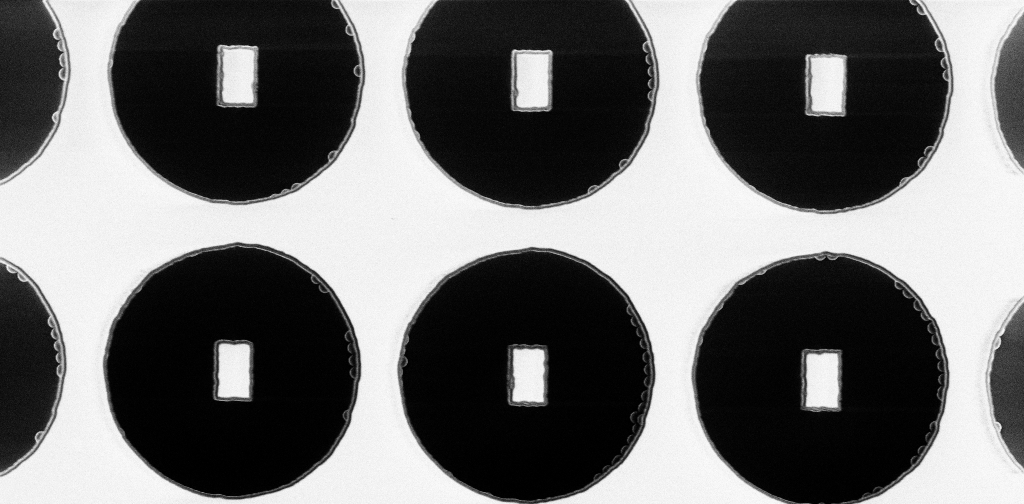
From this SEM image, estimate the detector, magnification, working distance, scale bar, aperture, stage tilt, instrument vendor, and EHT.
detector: InLens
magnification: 7.65 K X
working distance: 5.3 mm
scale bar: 2000 nm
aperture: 30 µm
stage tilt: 0°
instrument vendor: Zeiss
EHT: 3 kV